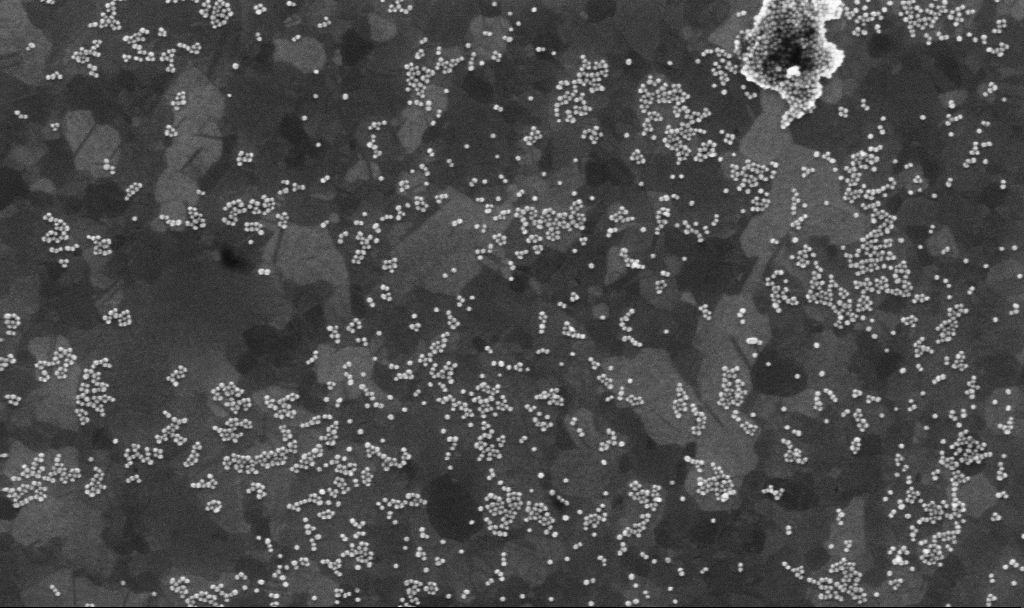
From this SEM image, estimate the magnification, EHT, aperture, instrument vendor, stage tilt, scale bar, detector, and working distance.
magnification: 100 K X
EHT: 10 kV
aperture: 30 µm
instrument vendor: Zeiss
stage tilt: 0°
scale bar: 200 nm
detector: InLens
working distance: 3.8 mm